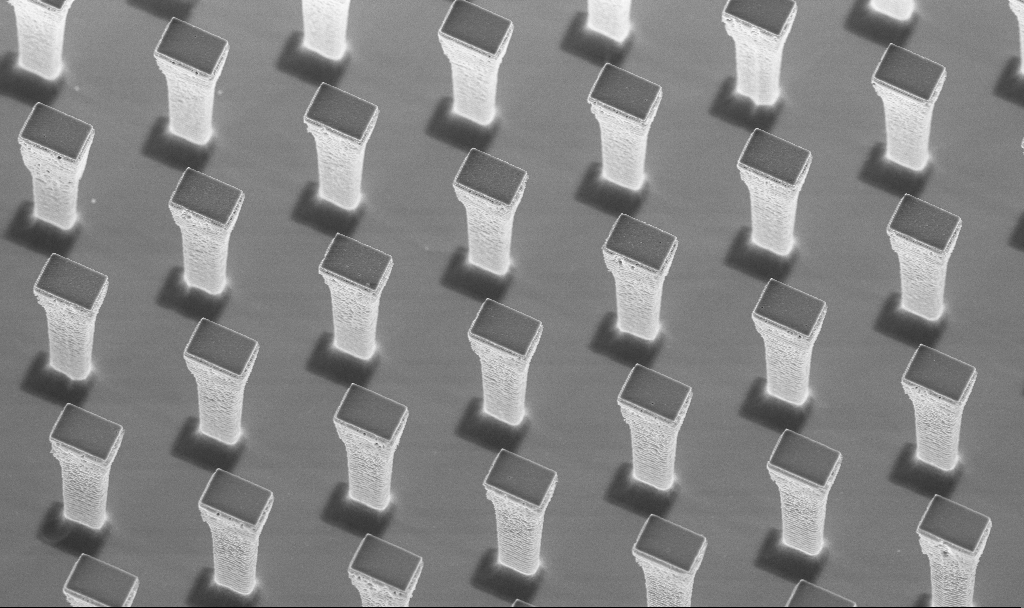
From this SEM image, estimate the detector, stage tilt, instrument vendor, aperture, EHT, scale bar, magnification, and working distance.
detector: InLens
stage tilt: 20°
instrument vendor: Zeiss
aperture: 30 µm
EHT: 5 kV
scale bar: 10000 nm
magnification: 5.07 K X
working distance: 4.2 mm